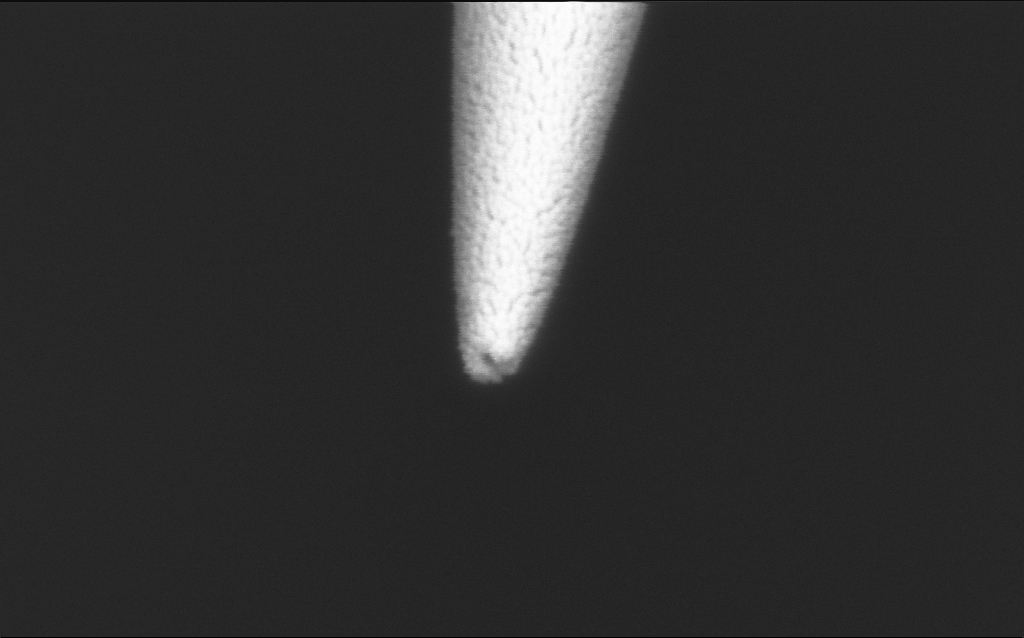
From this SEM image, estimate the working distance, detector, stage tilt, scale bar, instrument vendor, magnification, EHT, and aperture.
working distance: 7.6 mm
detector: InLens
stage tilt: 45°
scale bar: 200 nm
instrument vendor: Zeiss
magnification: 200 K X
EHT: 2 kV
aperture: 30 µm